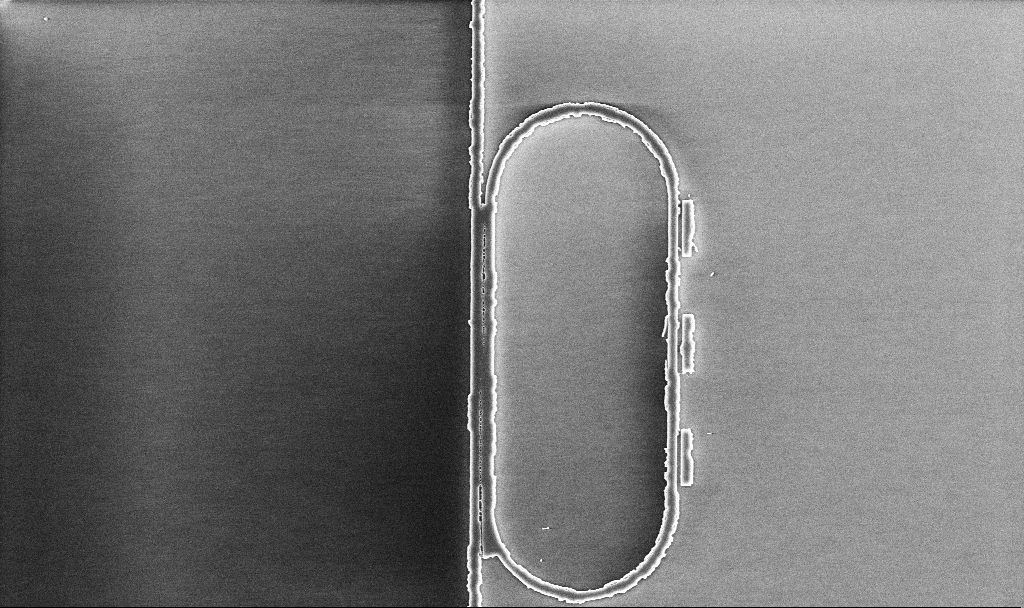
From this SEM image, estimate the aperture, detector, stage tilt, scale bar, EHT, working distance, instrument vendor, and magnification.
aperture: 30 µm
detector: InLens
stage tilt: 0°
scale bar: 10000 nm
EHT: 5 kV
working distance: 10.1 mm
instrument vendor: Zeiss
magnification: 7.13 K X